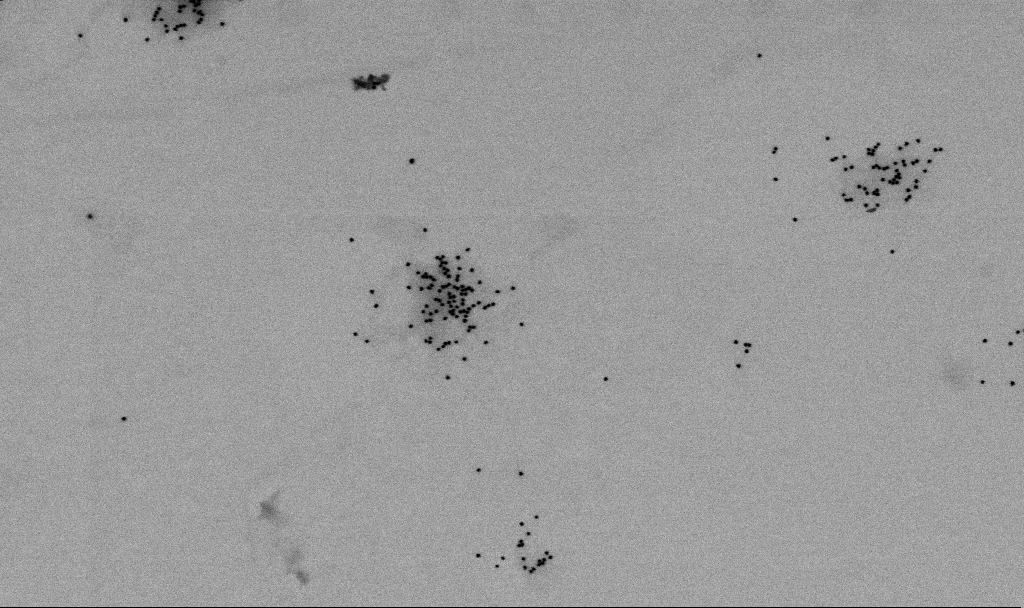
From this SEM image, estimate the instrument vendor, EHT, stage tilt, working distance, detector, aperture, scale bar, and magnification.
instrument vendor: Zeiss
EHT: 2 kV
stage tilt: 0°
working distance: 6.5 mm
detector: SE2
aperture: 30 µm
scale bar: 1000 nm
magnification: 60 K X